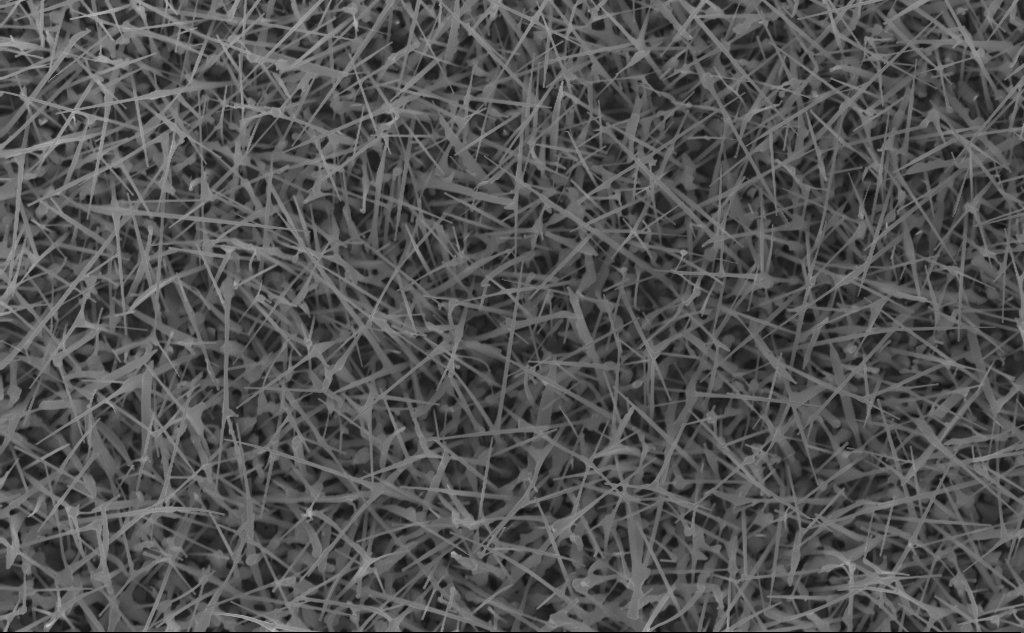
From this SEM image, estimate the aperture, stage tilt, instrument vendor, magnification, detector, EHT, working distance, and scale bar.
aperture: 30 µm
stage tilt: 0°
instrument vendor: Zeiss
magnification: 20 K X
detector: InLens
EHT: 10 kV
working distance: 5 mm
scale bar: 1000 nm